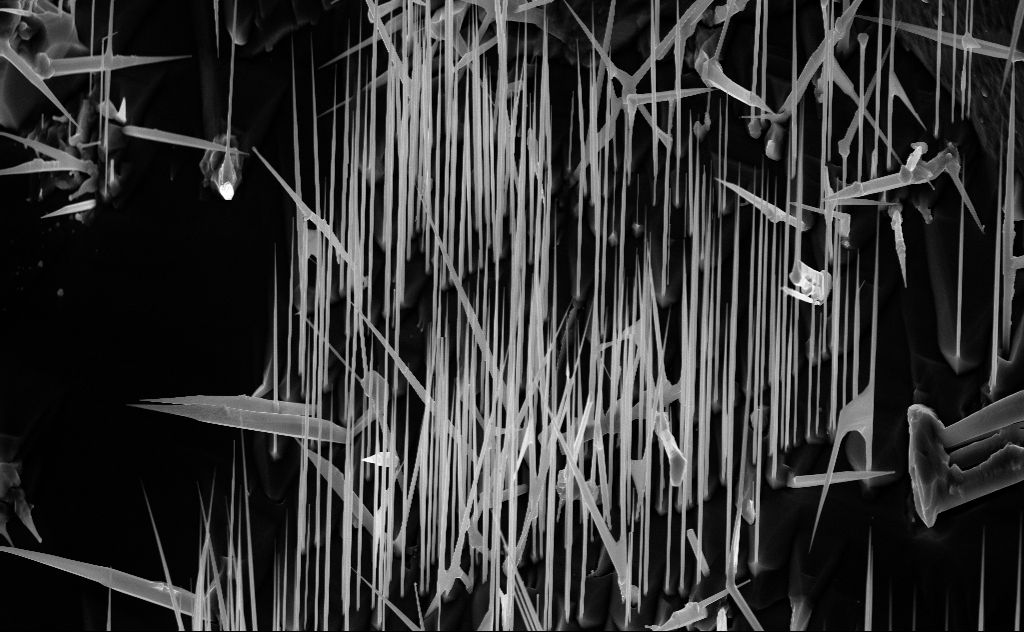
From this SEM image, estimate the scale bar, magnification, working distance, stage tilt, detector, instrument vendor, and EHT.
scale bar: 2000 nm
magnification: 10 K X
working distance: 7 mm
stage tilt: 44.9°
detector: InLens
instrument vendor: Zeiss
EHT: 10 kV